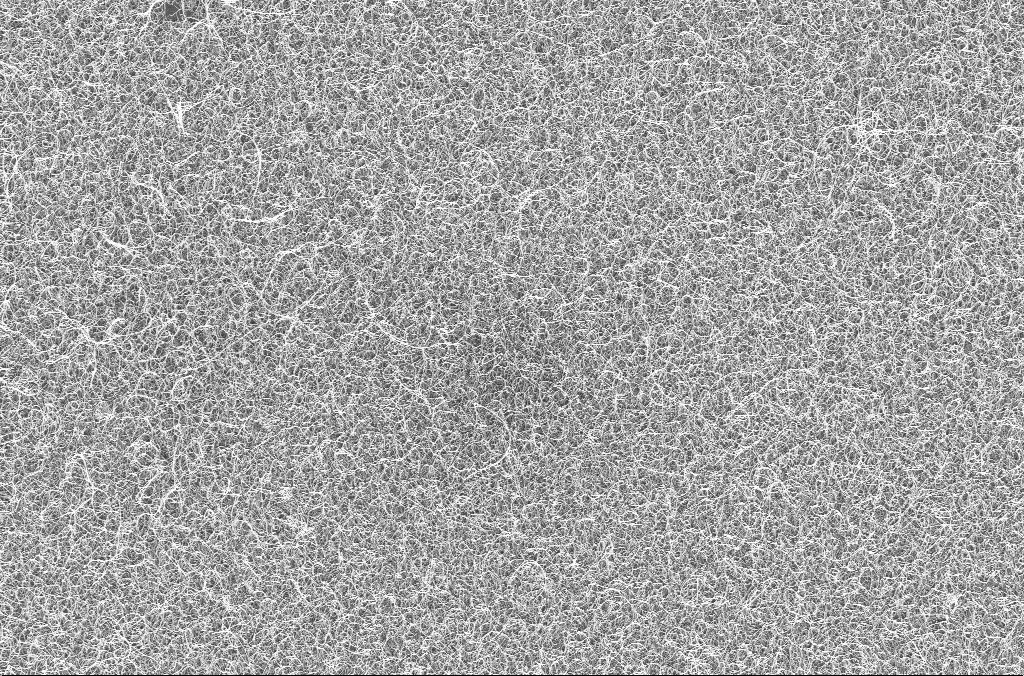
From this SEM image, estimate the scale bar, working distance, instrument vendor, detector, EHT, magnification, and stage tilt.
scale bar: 10000 nm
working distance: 4.5 mm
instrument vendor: Zeiss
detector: InLens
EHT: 20 kV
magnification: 5 K X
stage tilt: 0°